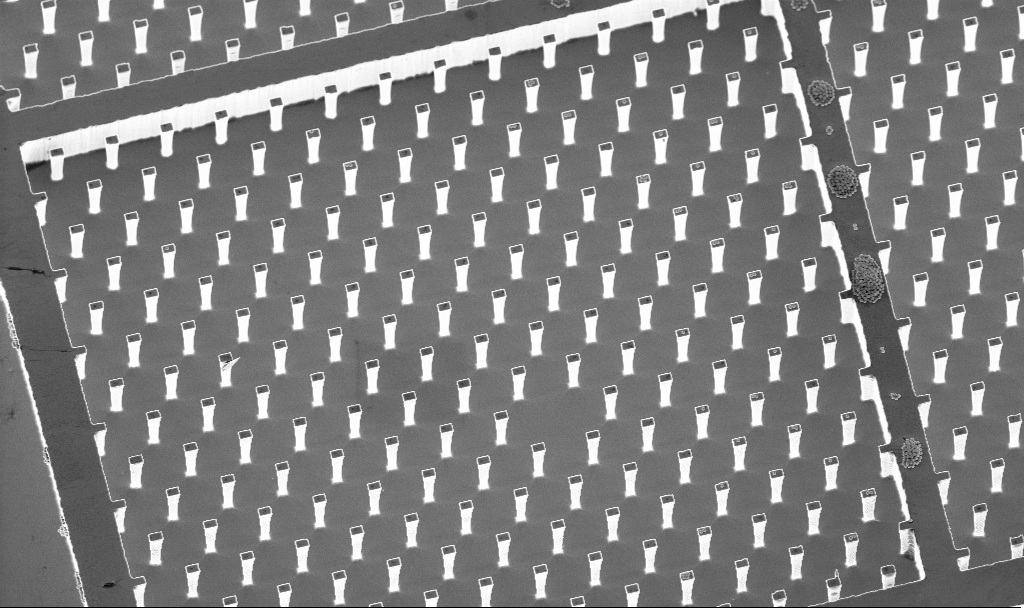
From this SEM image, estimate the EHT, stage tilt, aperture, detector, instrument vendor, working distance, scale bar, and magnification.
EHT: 5 kV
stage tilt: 20°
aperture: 30 µm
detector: InLens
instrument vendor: Zeiss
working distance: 4.7 mm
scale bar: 10000 nm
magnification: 1.73 K X